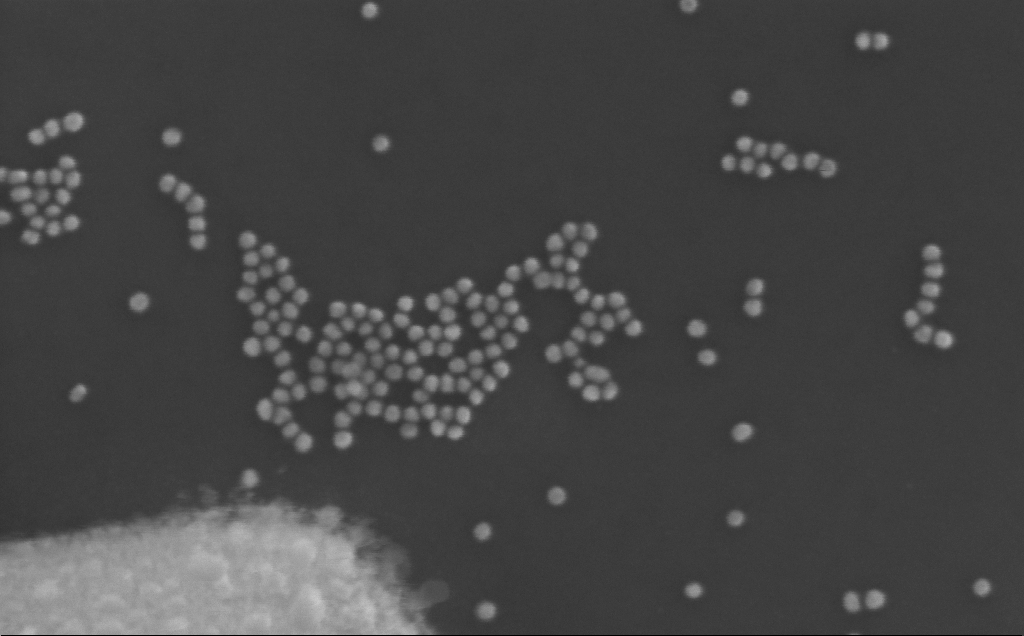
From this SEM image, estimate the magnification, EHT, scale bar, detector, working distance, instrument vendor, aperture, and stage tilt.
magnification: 376.5 K X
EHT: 10 kV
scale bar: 100 nm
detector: InLens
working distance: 3 mm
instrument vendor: Zeiss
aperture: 30 µm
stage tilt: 0°